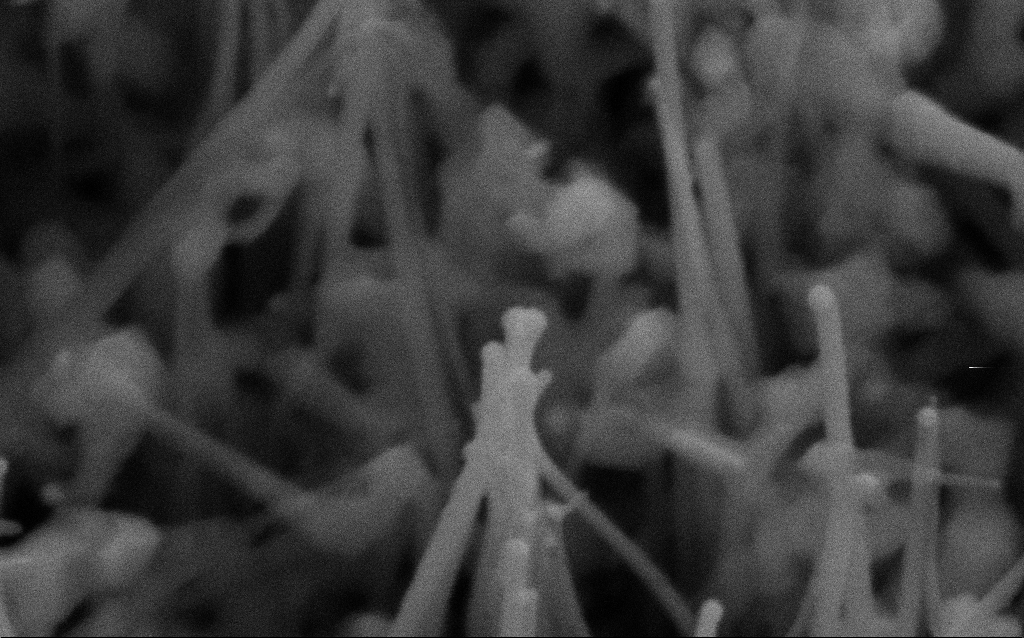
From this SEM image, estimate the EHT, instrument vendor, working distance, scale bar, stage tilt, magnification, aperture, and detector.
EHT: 10 kV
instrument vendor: Zeiss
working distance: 7.3 mm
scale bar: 100 nm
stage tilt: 35°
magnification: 303.78 K X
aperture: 30 µm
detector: SE2